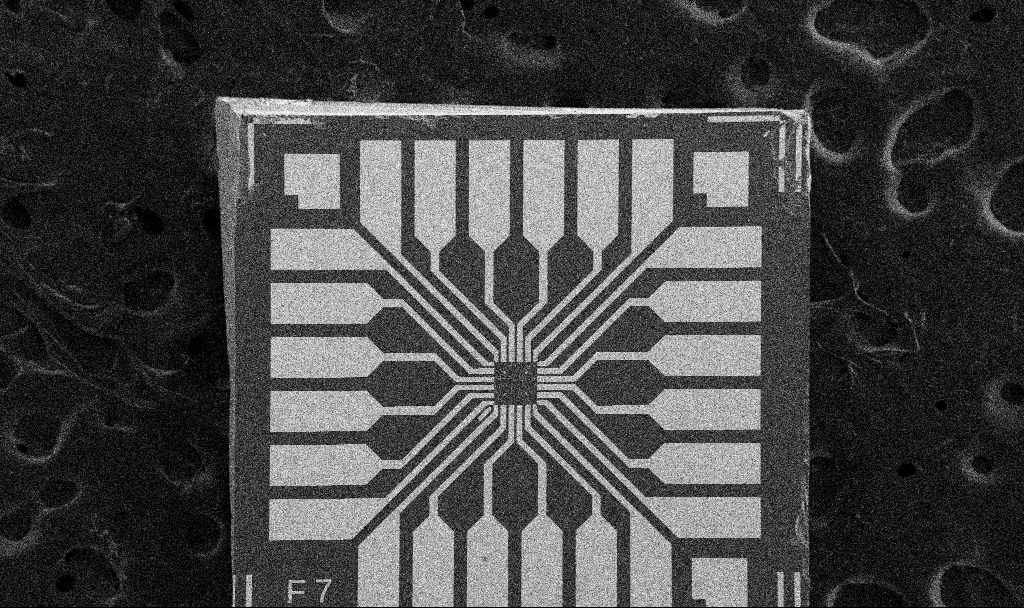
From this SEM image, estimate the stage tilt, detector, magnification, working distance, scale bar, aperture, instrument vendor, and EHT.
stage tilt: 0°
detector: SE2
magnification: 0.1 K X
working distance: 8.7 mm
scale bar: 200000 nm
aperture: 30 µm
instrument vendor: Zeiss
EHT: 5 kV